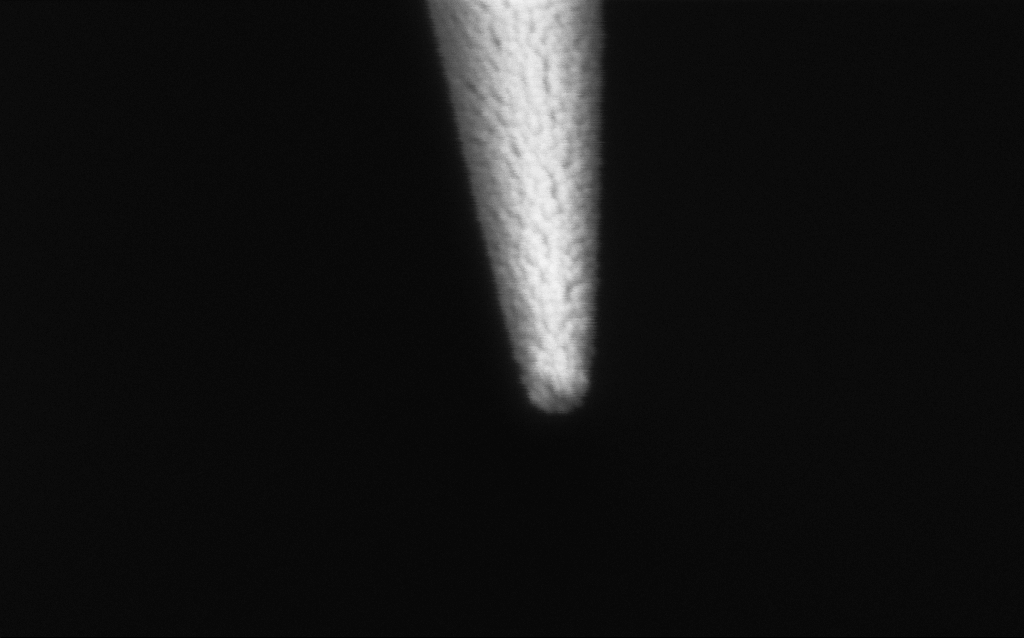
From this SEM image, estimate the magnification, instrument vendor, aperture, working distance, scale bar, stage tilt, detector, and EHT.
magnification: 250 K X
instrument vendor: Zeiss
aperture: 30 µm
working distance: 7.7 mm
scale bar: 200 nm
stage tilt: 45°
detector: InLens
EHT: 2 kV